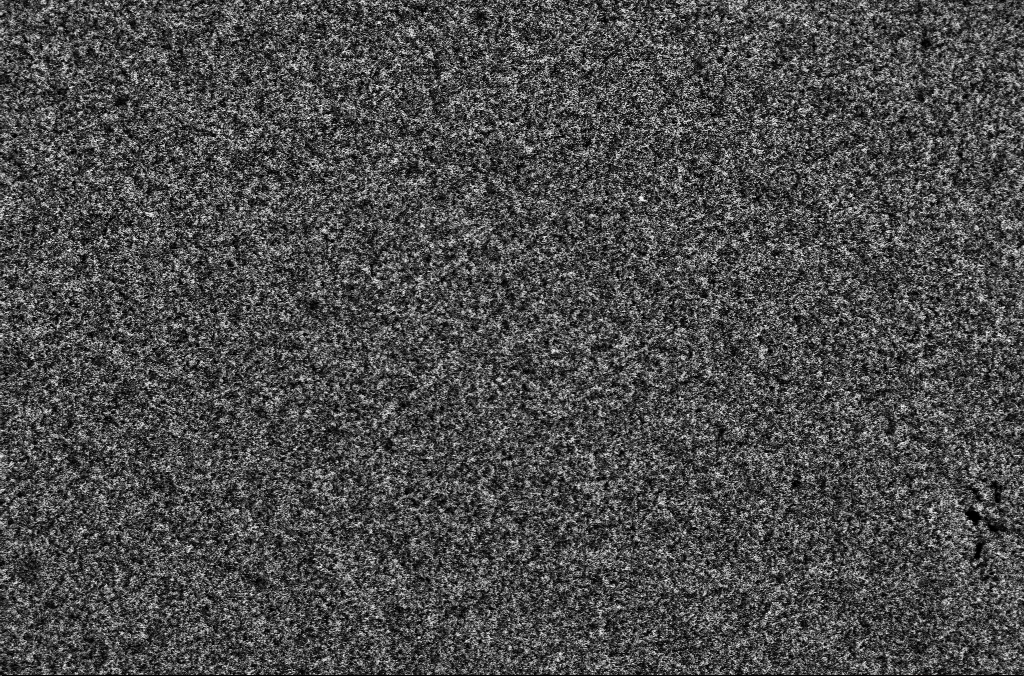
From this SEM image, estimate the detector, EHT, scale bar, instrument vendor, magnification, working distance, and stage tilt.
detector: SE2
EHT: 1.8 kV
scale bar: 20000 nm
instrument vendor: Zeiss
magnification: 1 K X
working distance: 5.3 mm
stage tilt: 0°